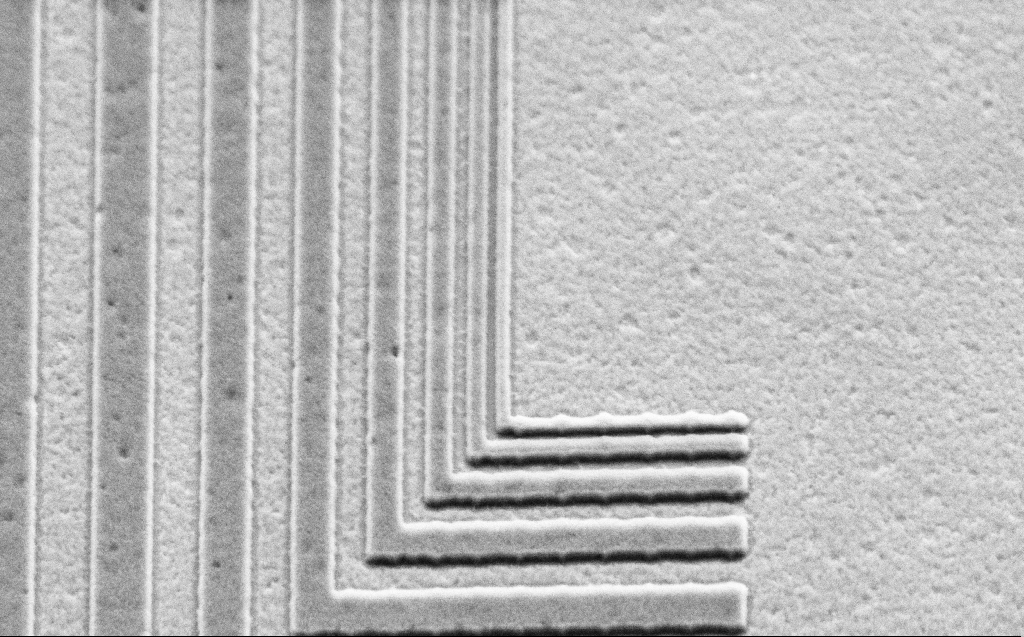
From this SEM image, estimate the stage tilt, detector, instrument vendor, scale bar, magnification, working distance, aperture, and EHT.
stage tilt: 44.1°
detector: SE2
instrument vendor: Zeiss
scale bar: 2000 nm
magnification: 30.85 K X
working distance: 6 mm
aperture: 30 µm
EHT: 2.5 kV